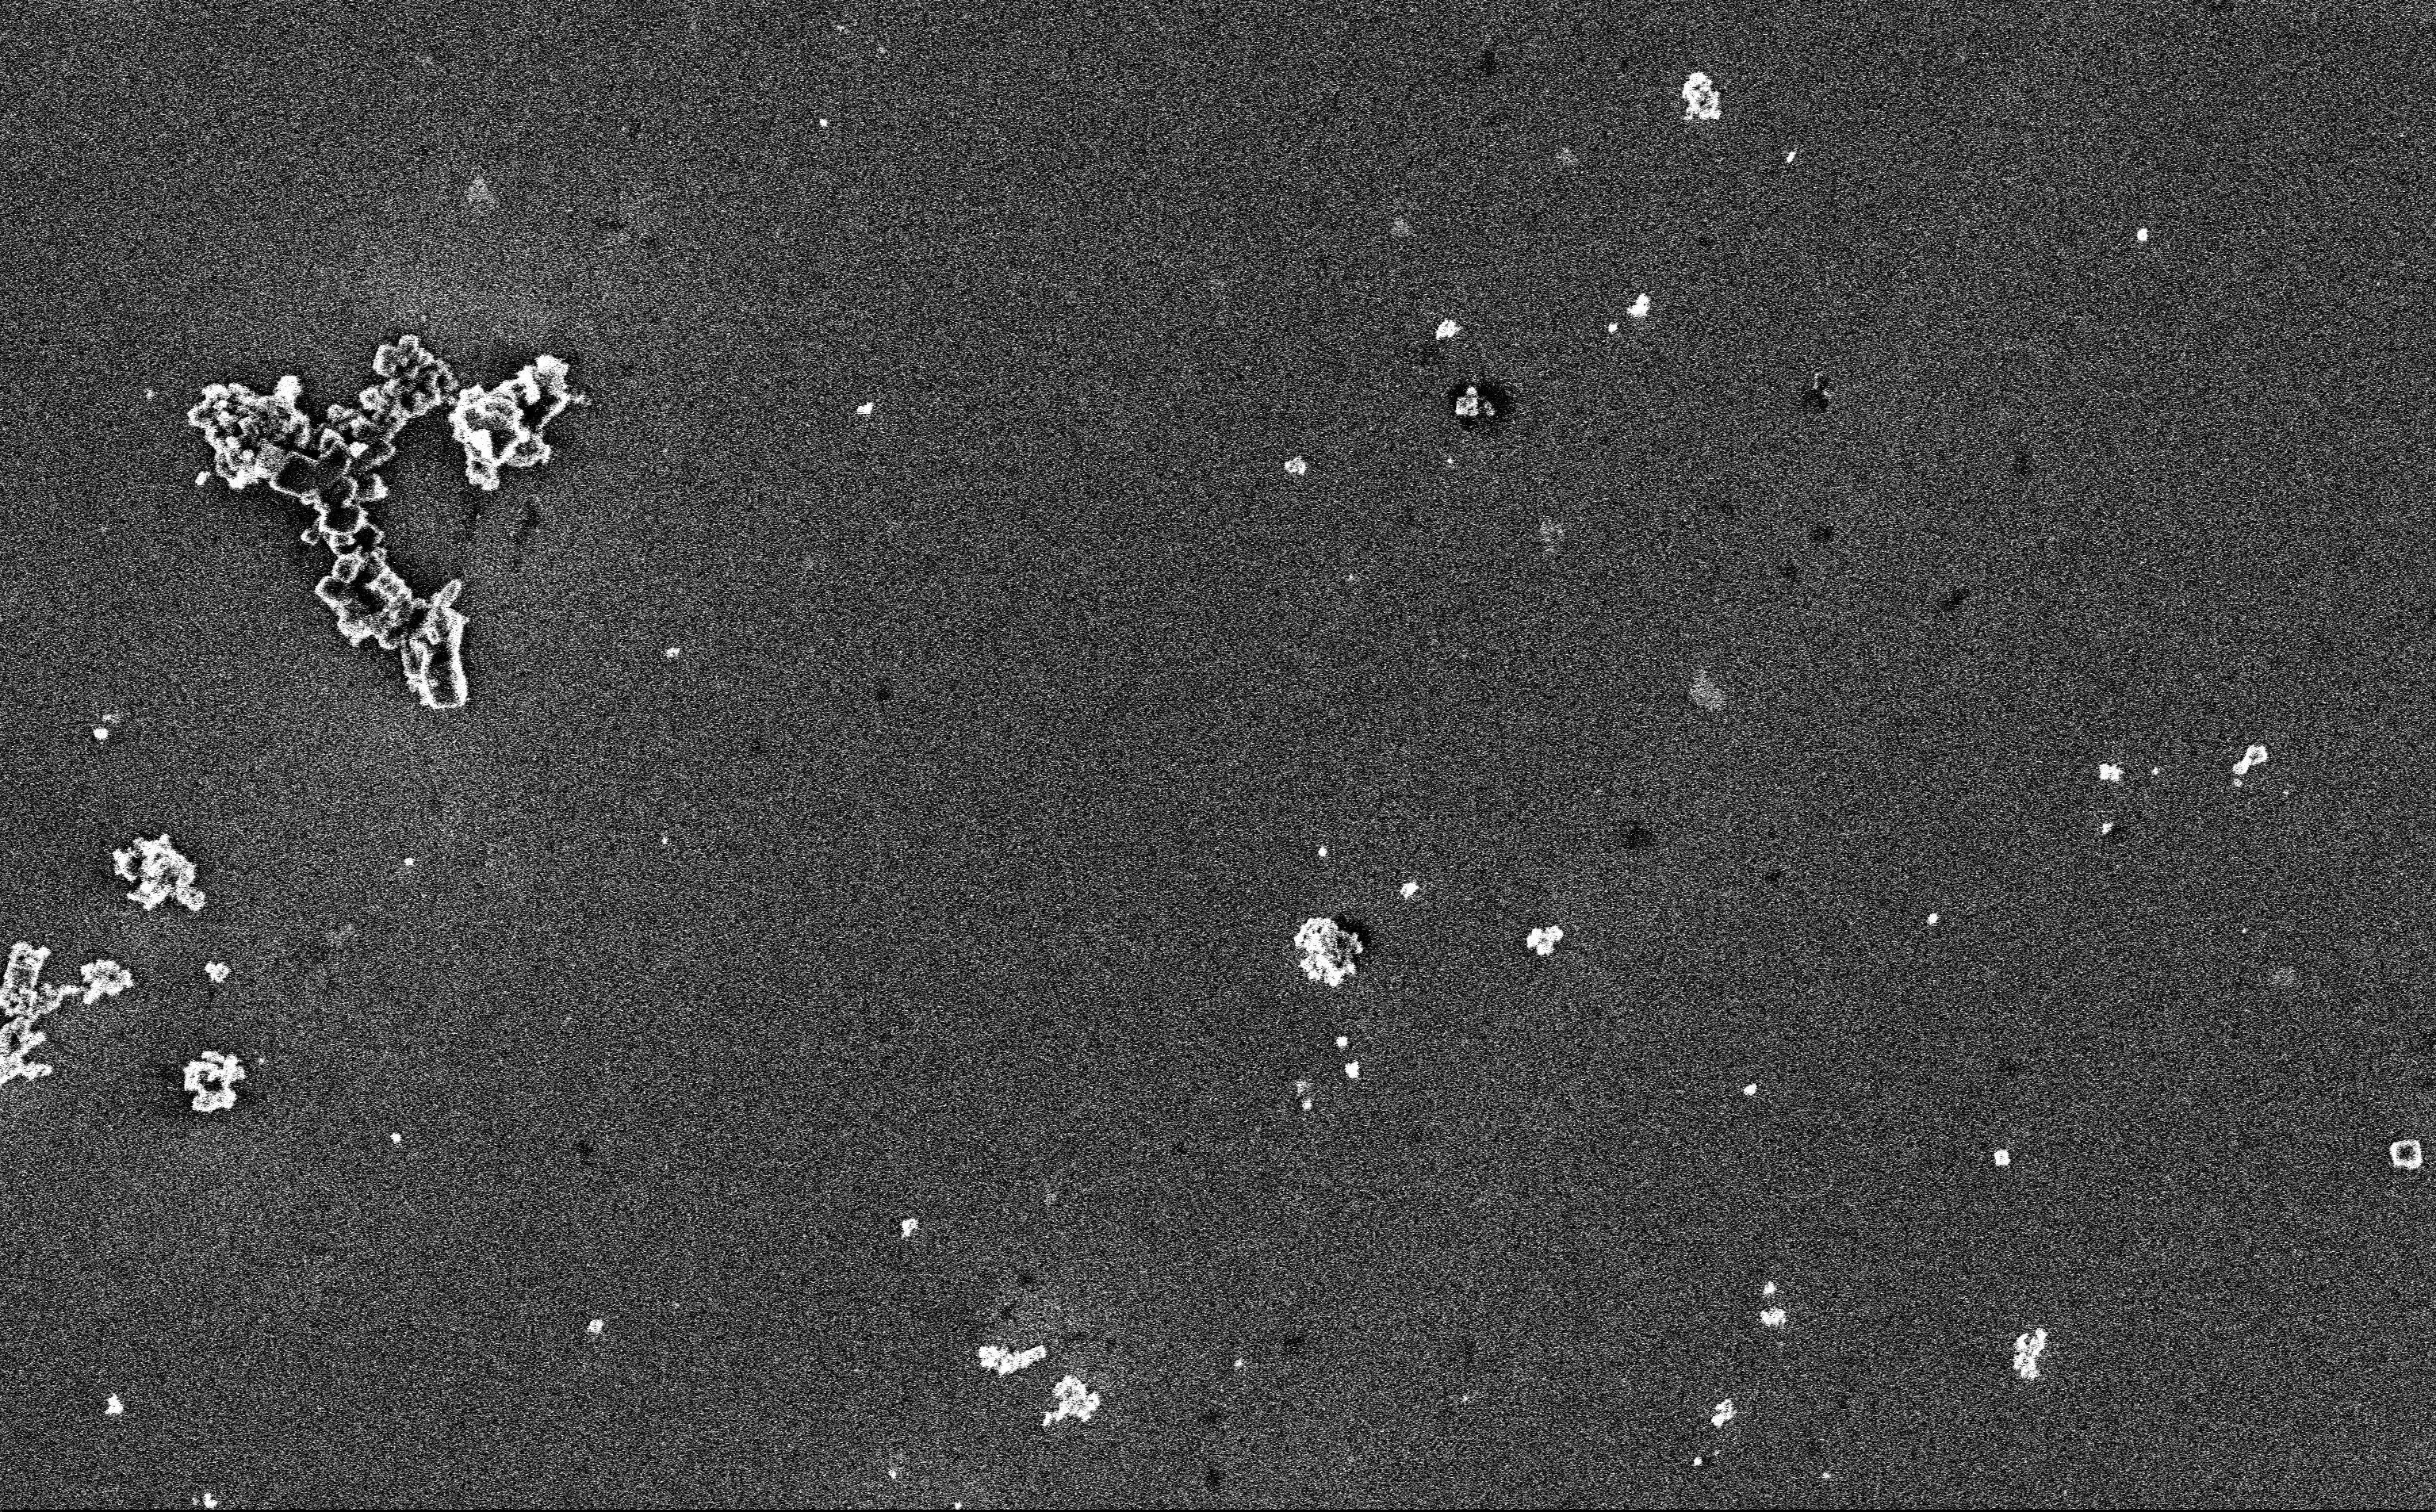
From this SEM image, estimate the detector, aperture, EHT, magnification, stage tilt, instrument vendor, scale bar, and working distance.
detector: InLens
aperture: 30 µm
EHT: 3 kV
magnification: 9.98 K X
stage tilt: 0°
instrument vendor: Zeiss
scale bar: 2000 nm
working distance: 3 mm